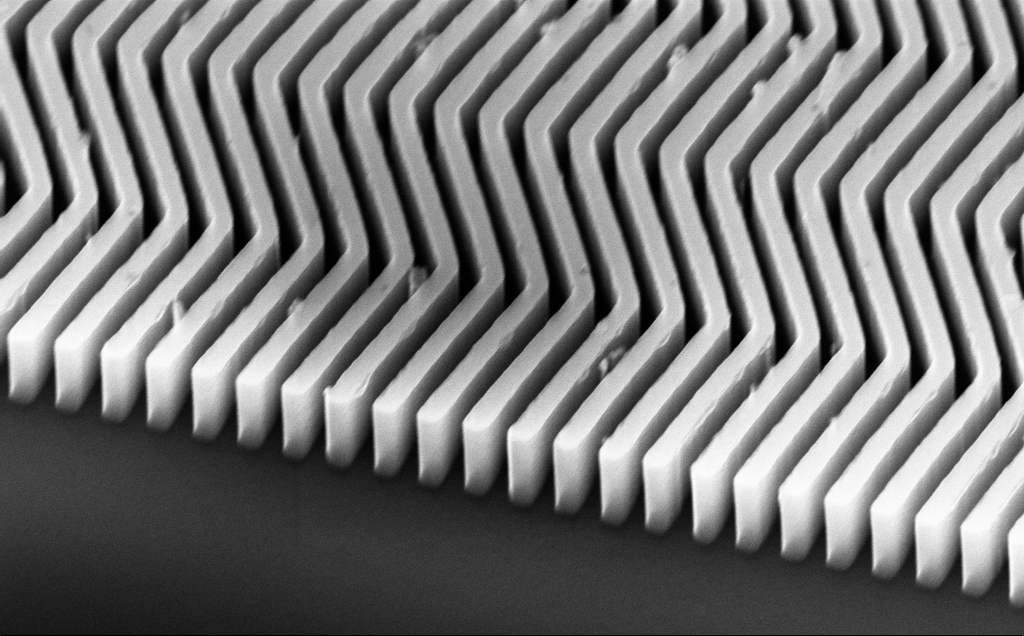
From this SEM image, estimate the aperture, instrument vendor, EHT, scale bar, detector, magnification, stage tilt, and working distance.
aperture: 30 µm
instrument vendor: Zeiss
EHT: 10 kV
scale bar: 1000 nm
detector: InLens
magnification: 56.65 K X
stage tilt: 45°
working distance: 6 mm